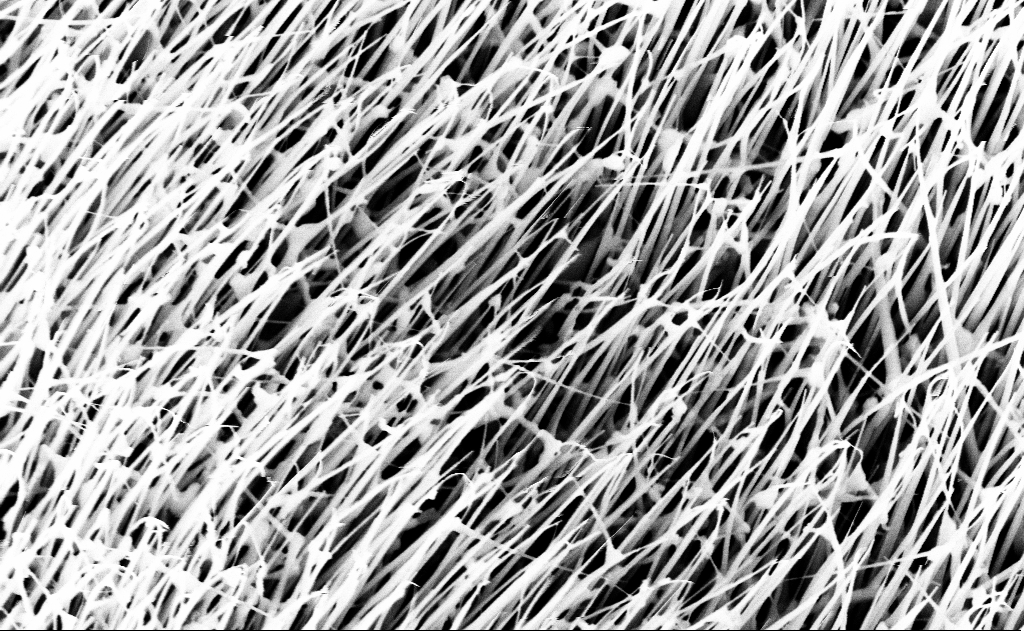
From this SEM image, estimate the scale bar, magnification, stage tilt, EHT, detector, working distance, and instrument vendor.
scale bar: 1000 nm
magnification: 40 K X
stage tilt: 0°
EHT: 10 kV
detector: InLens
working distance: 13 mm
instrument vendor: Zeiss